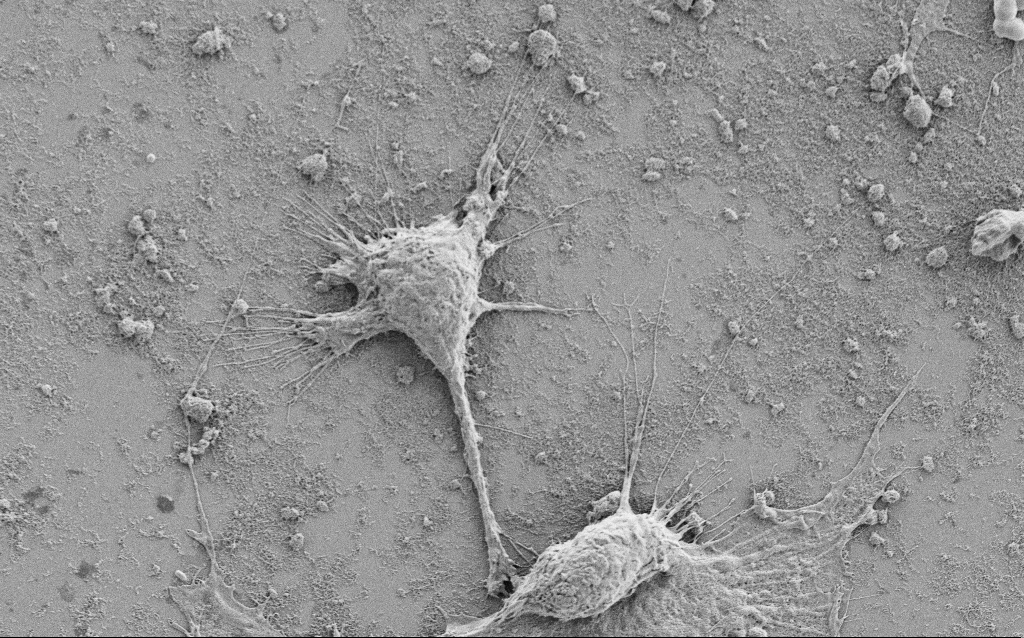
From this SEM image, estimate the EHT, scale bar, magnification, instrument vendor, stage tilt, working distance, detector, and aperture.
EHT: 1.5 kV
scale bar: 10000 nm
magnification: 3.5 K X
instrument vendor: Zeiss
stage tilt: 0°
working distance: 6.9 mm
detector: SE2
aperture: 30 µm